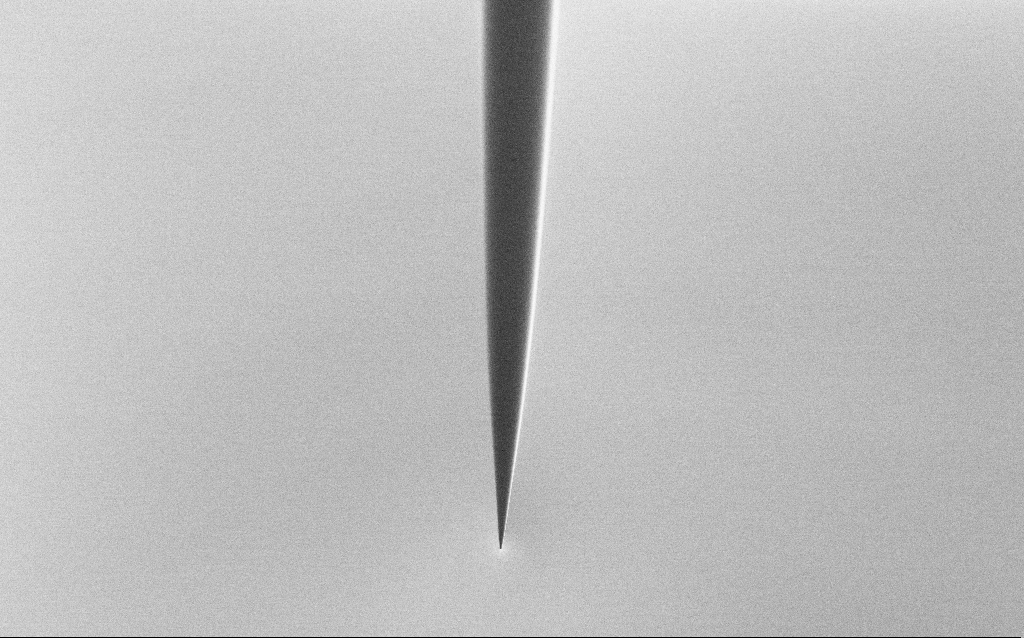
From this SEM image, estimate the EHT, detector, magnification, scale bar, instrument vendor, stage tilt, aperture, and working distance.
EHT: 2 kV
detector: SE2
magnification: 1 K X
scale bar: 20000 nm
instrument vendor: Zeiss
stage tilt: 45°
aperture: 30 µm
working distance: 6 mm